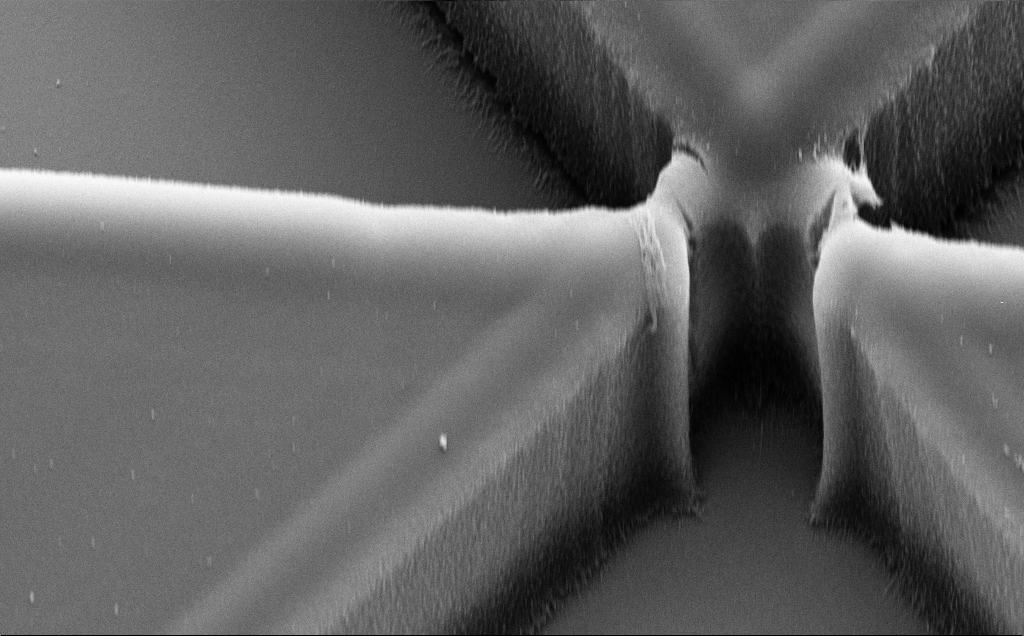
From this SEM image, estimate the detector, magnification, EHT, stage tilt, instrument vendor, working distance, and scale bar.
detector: SE2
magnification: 8.78 K X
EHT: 10 kV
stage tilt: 35.3°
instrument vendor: Zeiss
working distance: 11 mm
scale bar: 2000 nm